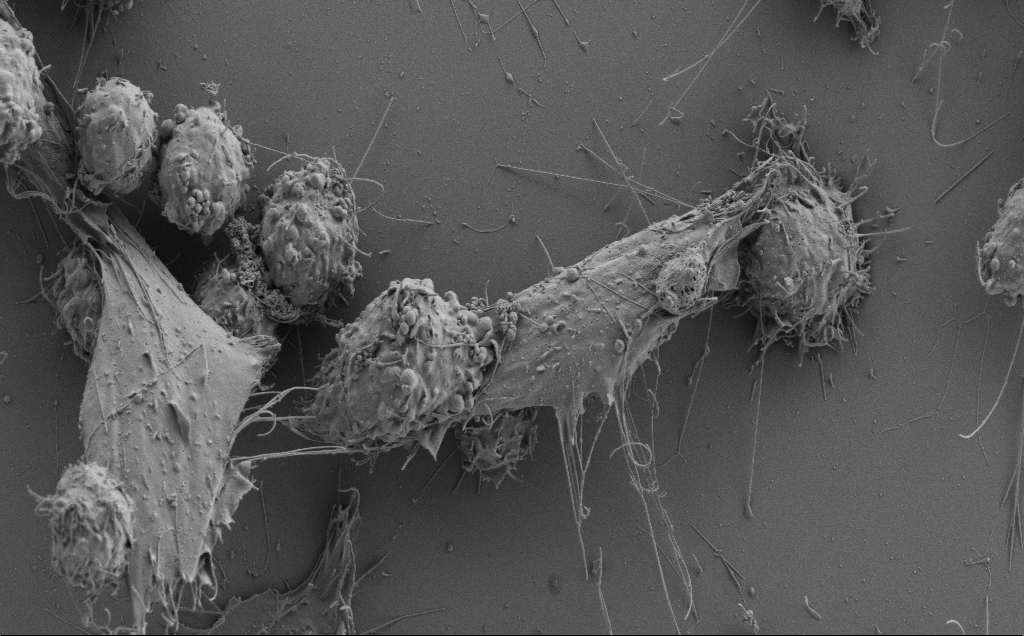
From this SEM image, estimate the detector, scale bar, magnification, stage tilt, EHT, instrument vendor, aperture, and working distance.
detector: SE2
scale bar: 10000 nm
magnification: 4.3 K X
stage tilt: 0.8°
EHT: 3 kV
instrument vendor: Zeiss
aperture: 30 µm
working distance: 6 mm